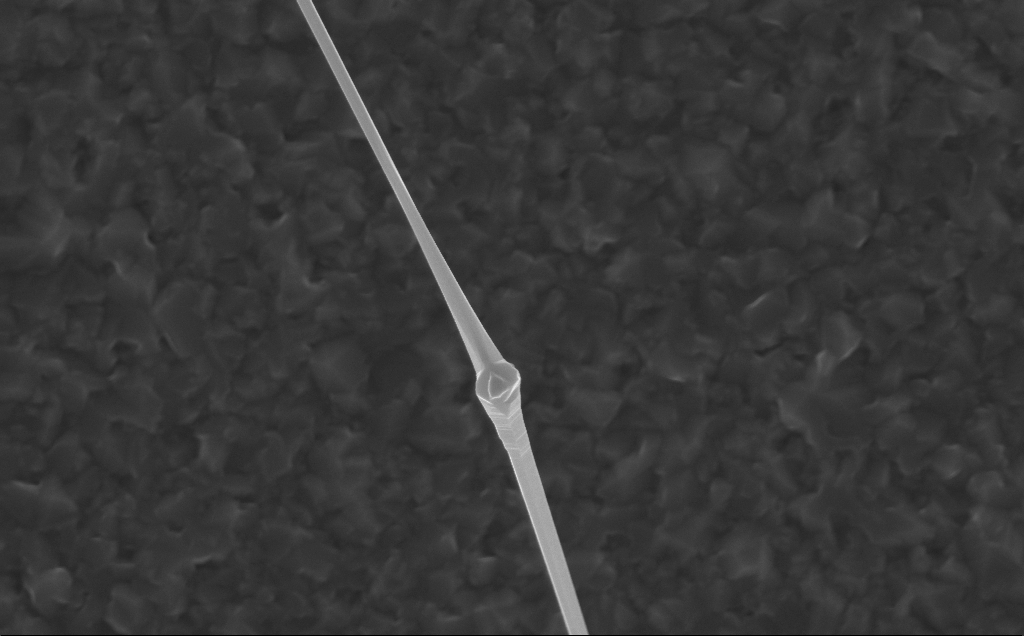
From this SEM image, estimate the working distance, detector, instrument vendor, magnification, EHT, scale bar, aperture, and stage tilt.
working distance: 5 mm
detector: InLens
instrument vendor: Zeiss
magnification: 40 K X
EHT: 10 kV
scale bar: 1000 nm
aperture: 30 µm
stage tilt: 0°